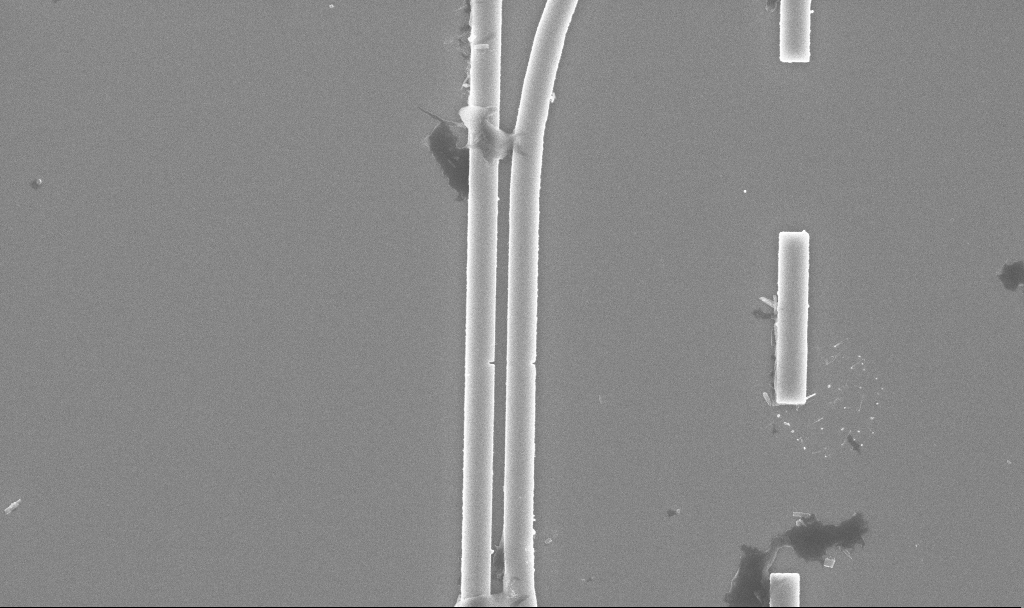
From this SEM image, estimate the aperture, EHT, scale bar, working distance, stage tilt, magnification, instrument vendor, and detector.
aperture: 30 µm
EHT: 5 kV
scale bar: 2000 nm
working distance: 9.9 mm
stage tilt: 0°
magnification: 21.1 K X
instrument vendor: Zeiss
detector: InLens